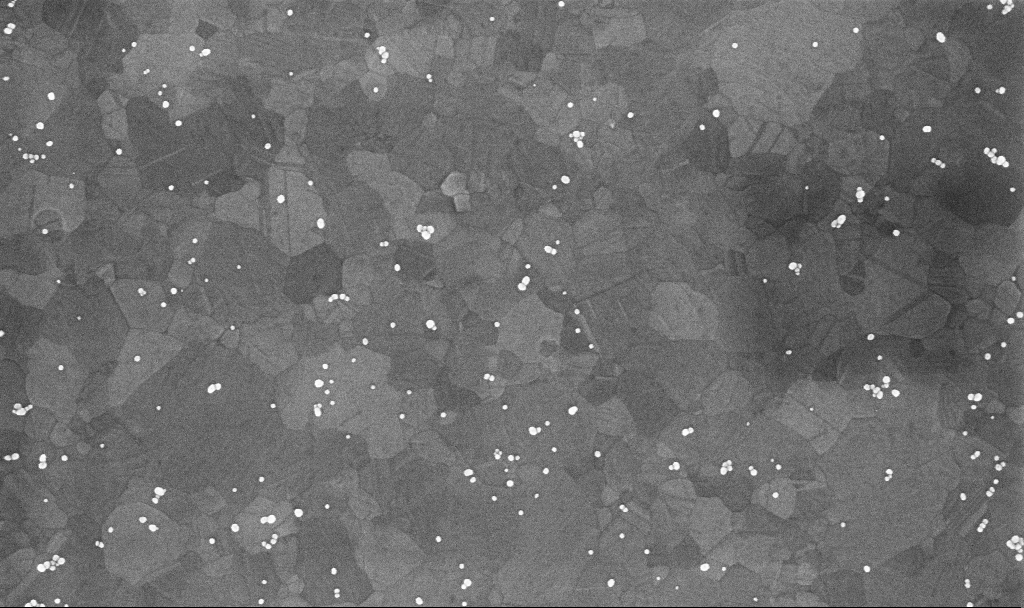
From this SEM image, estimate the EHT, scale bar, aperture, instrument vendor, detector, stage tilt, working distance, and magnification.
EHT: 10 kV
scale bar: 200 nm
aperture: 30 µm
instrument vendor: Zeiss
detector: InLens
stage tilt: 0°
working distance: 3.4 mm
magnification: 100.84 K X